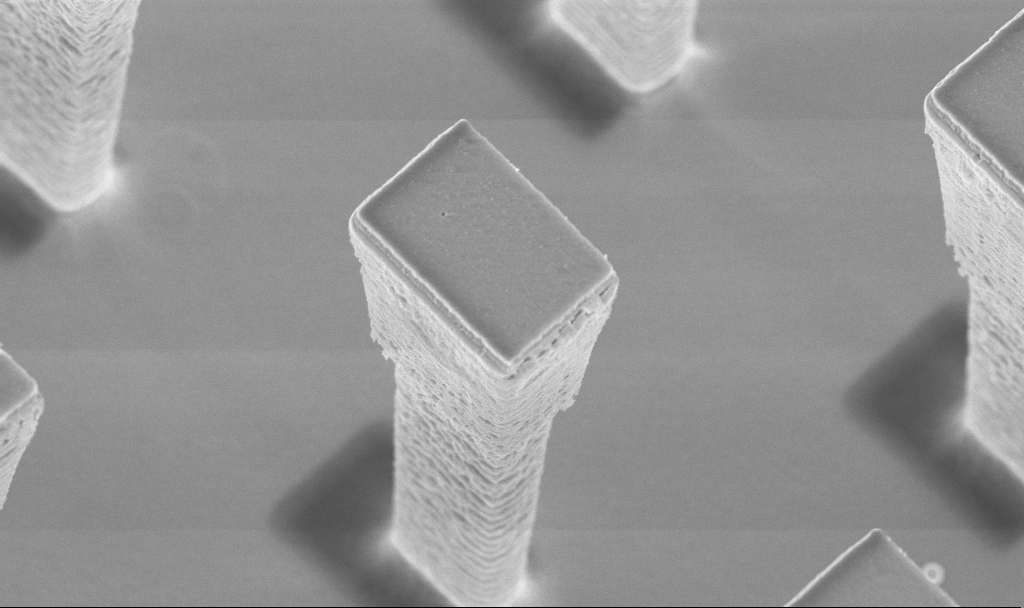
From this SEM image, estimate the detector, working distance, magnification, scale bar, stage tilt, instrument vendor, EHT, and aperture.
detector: InLens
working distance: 4.2 mm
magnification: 18.42 K X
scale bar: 1000 nm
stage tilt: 20°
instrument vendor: Zeiss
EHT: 5 kV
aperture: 30 µm